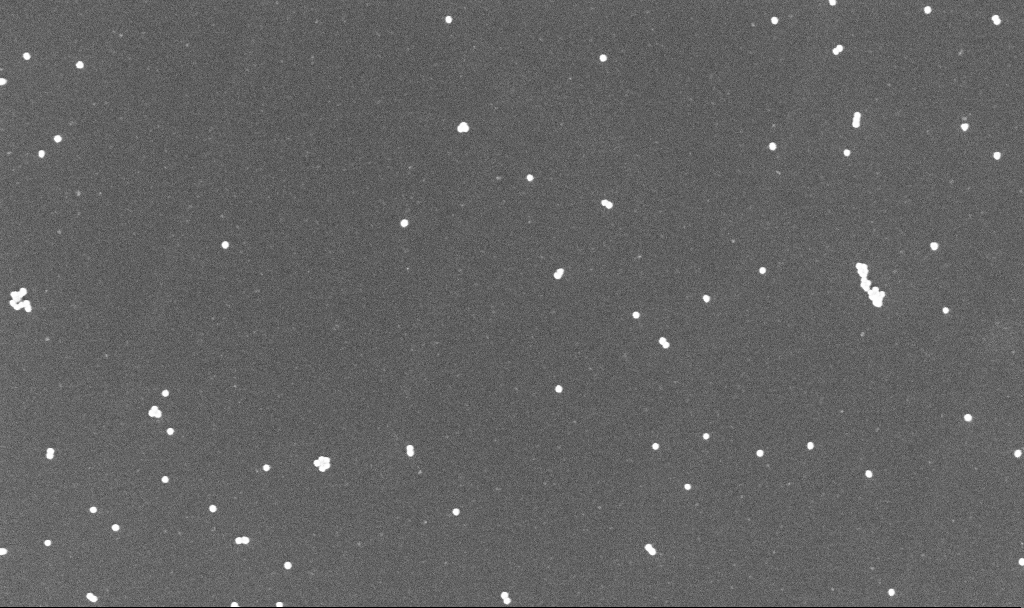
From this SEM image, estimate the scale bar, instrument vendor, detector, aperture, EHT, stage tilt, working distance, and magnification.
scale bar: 200 nm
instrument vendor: Zeiss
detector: InLens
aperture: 30 µm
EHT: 10 kV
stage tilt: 0°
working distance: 3.3 mm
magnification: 100 K X